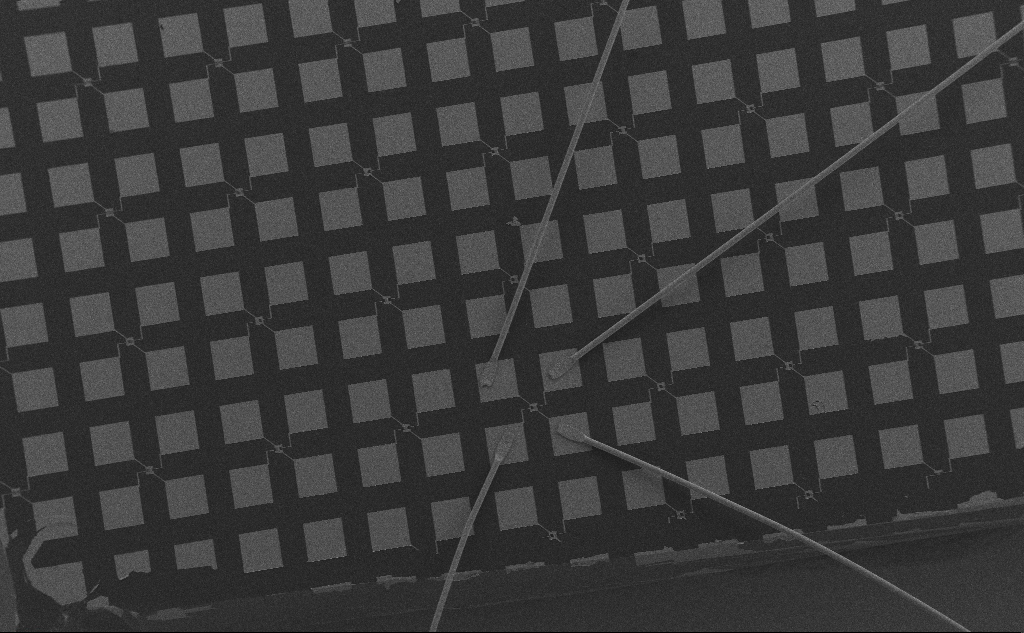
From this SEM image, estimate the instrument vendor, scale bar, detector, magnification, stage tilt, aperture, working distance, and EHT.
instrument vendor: Zeiss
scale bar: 200000 nm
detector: SE2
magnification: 0.096 K X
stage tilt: -0.1°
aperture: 30 µm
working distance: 6 mm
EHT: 10 kV